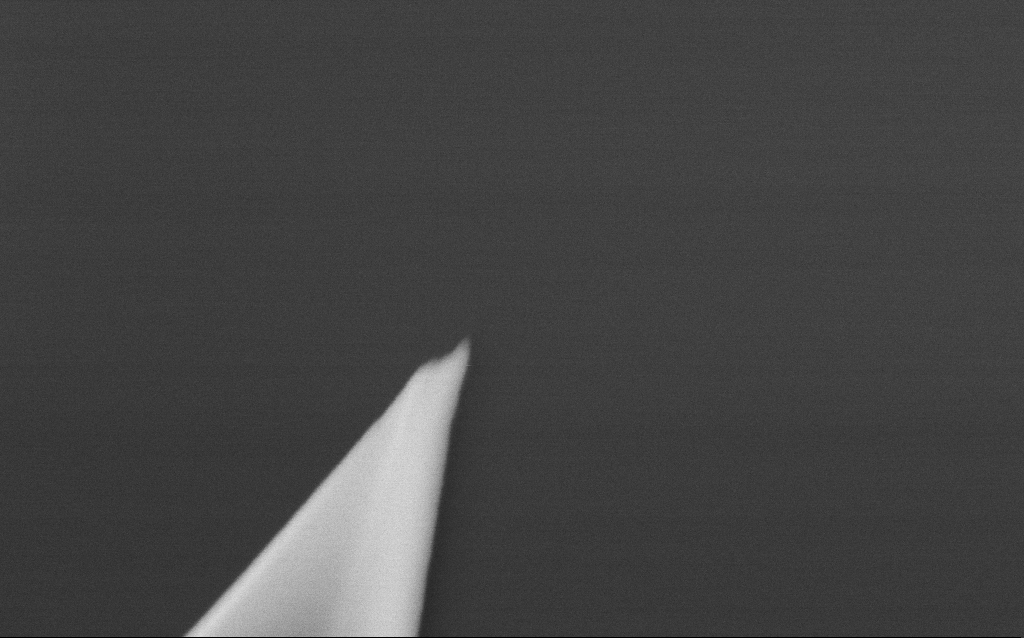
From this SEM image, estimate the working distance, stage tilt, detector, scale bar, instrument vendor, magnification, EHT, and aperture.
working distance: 5.2 mm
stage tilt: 0°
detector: InLens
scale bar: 100 nm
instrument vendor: Zeiss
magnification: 250 K X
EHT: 5 kV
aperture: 30 µm